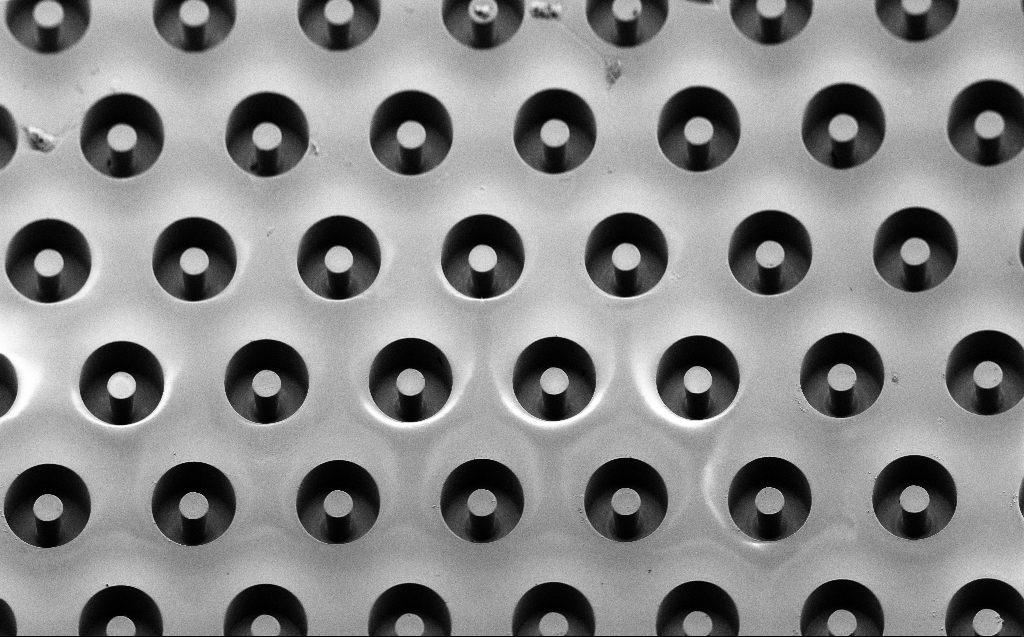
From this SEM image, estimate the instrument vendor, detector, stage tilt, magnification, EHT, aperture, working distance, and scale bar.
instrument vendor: Zeiss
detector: SE2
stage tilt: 45°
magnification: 0.496 K X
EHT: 2 kV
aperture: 30 µm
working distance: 6 mm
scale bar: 100000 nm